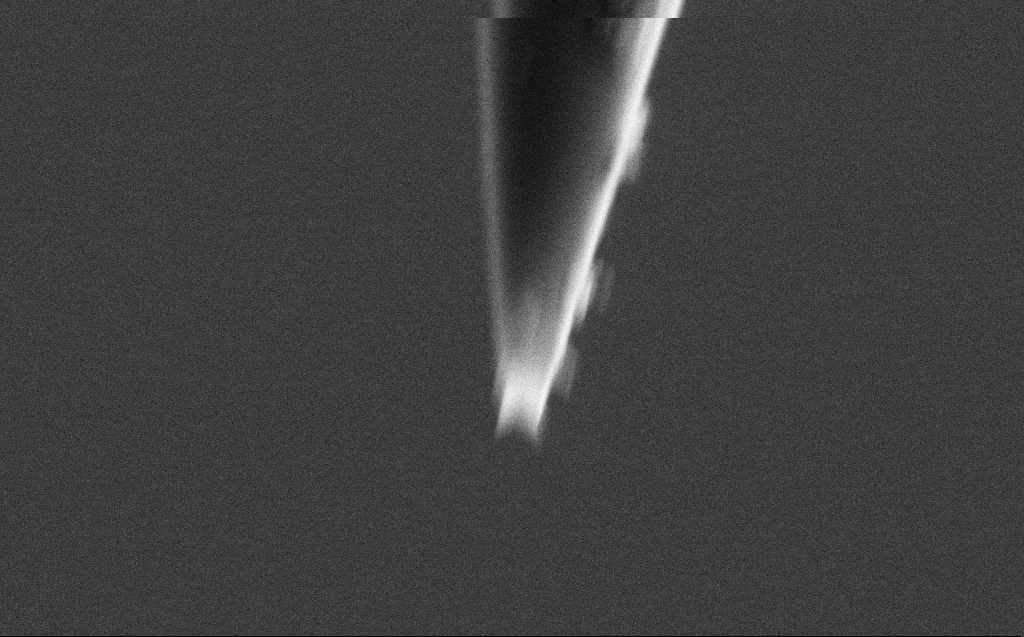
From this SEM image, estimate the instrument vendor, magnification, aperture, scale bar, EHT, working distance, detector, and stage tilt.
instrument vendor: Zeiss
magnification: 86.18 K X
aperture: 30 µm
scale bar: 200 nm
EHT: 2 kV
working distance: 4 mm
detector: SE2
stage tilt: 45°